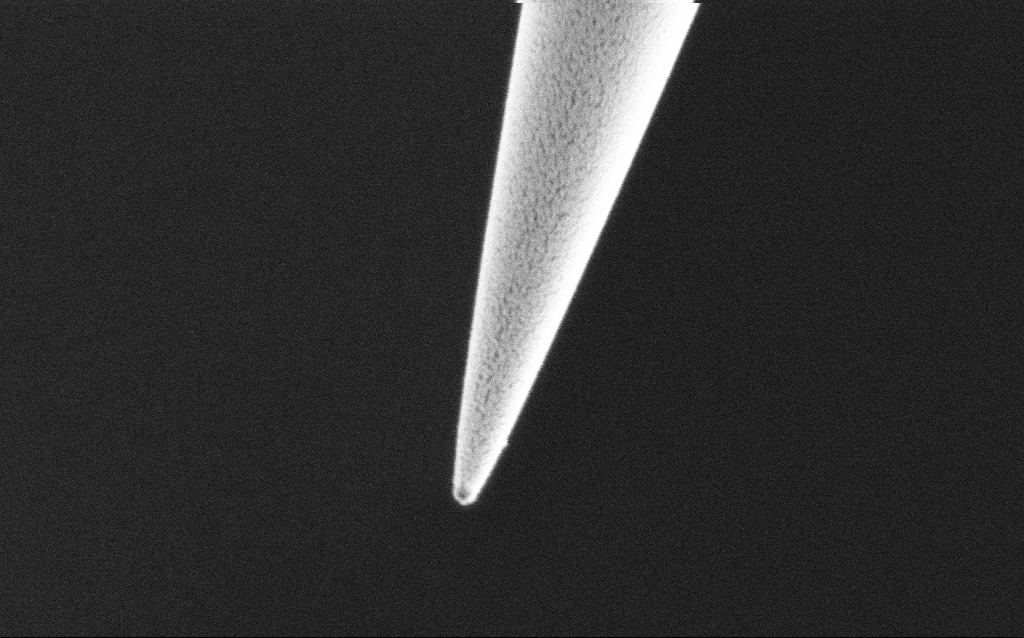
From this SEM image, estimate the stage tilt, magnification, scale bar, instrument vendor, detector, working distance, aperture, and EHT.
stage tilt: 45°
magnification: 75 K X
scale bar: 200 nm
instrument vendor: Zeiss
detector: SE2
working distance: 7.6 mm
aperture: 30 µm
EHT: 2 kV